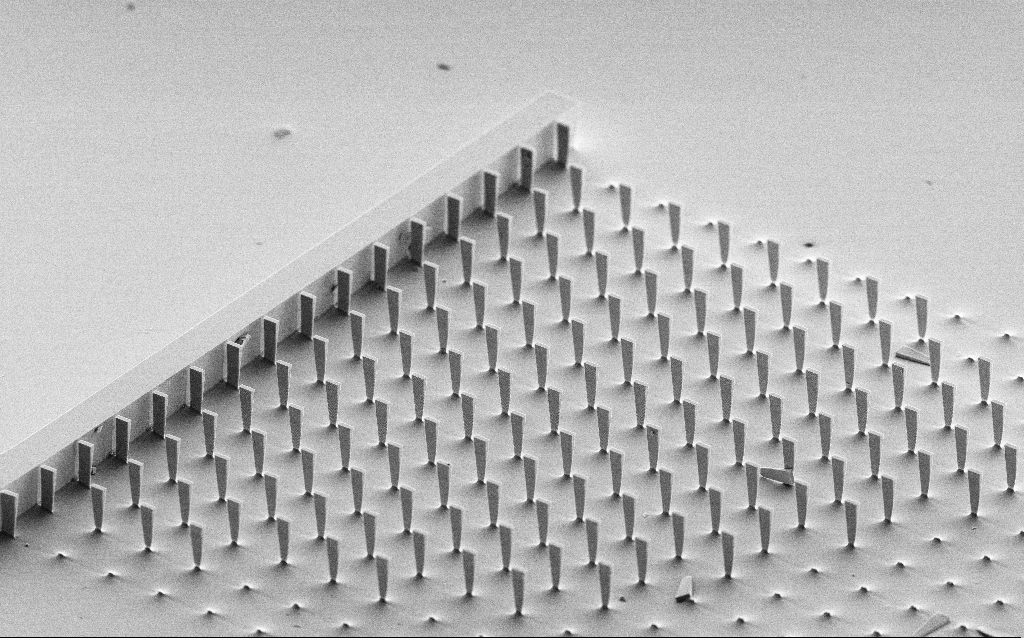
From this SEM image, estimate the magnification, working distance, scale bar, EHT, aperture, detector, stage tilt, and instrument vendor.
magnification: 0.754 K X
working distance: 9.7 mm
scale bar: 20000 nm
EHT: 5 kV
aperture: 30 µm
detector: SE2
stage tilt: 60°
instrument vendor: Zeiss